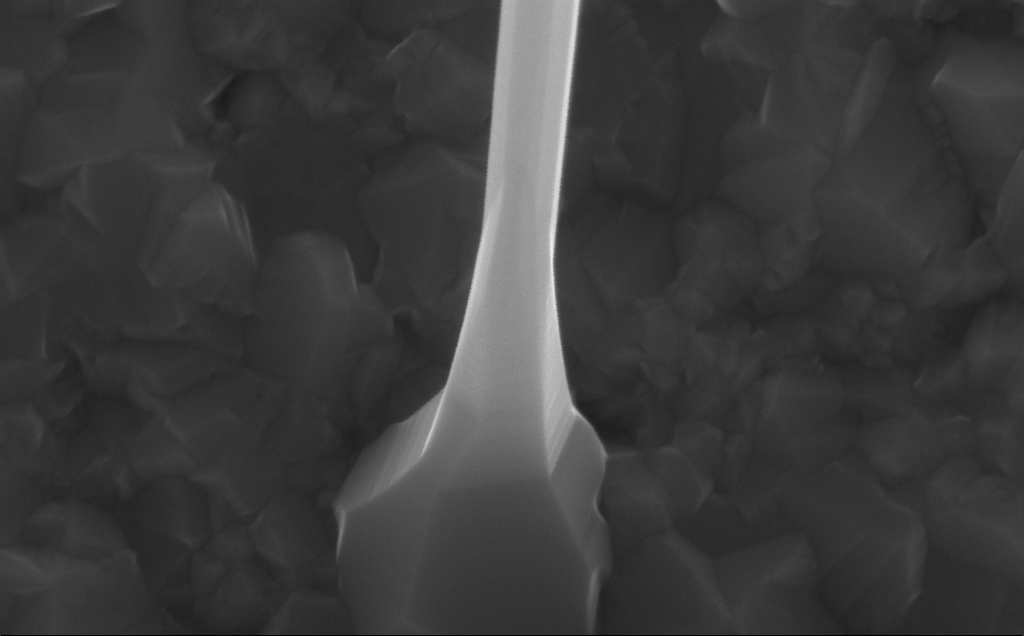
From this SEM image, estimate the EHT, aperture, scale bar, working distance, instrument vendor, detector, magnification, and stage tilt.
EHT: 10 kV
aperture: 30 µm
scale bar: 200 nm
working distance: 5 mm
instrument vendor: Zeiss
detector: InLens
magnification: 162.8 K X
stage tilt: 0°